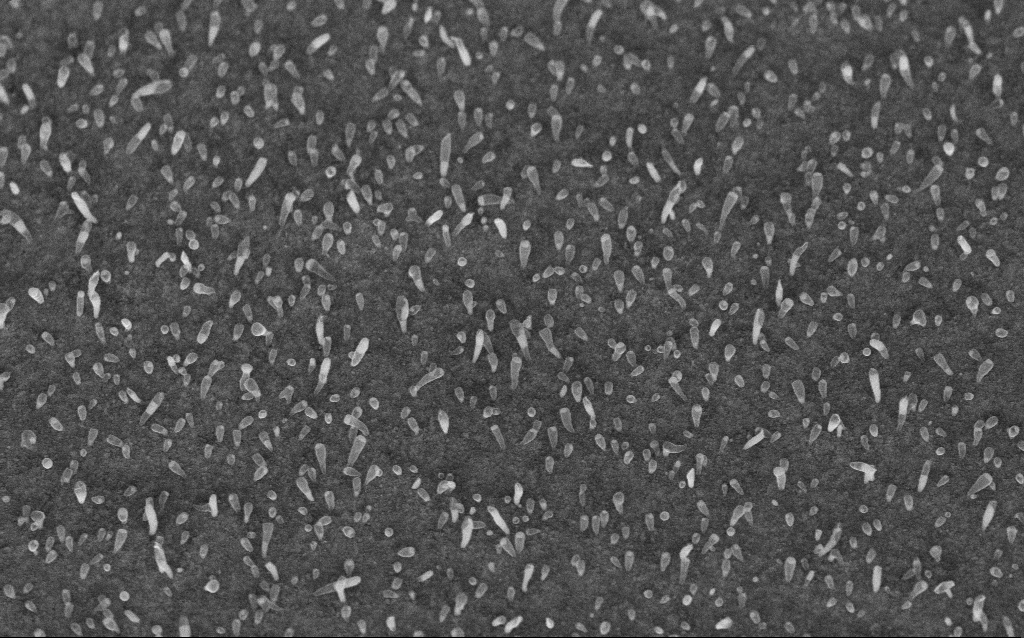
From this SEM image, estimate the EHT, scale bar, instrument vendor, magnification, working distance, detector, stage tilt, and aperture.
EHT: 5 kV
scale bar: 1000 nm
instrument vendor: Zeiss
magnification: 50 K X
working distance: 7.2 mm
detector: InLens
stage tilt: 45°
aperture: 30 µm